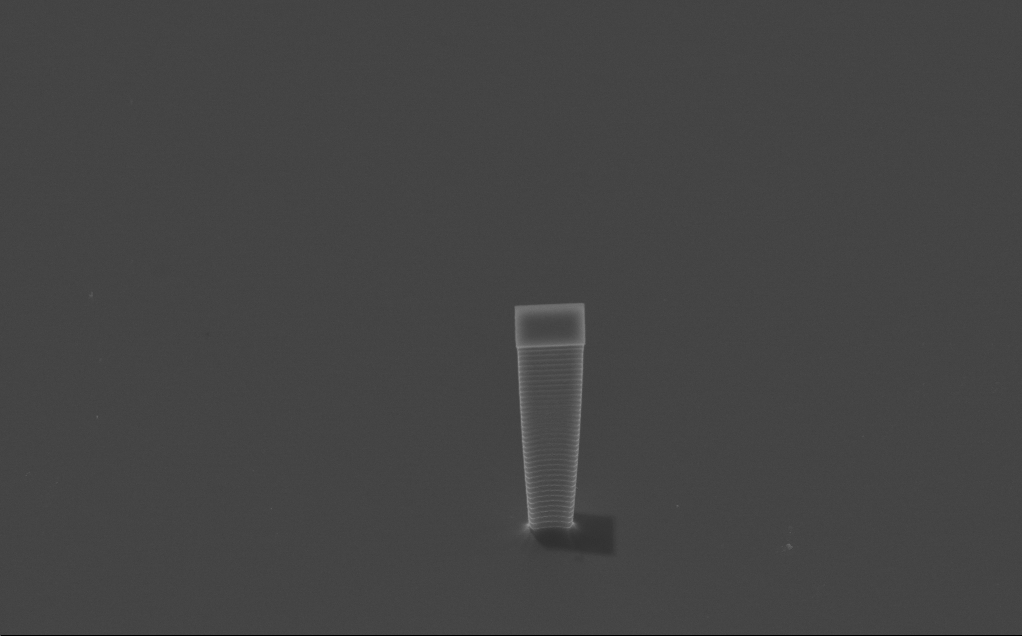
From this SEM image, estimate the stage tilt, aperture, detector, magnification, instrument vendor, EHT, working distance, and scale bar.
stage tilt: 45°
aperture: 30 µm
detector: InLens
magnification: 5.27 K X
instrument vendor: Zeiss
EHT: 10 kV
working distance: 4 mm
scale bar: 10000 nm